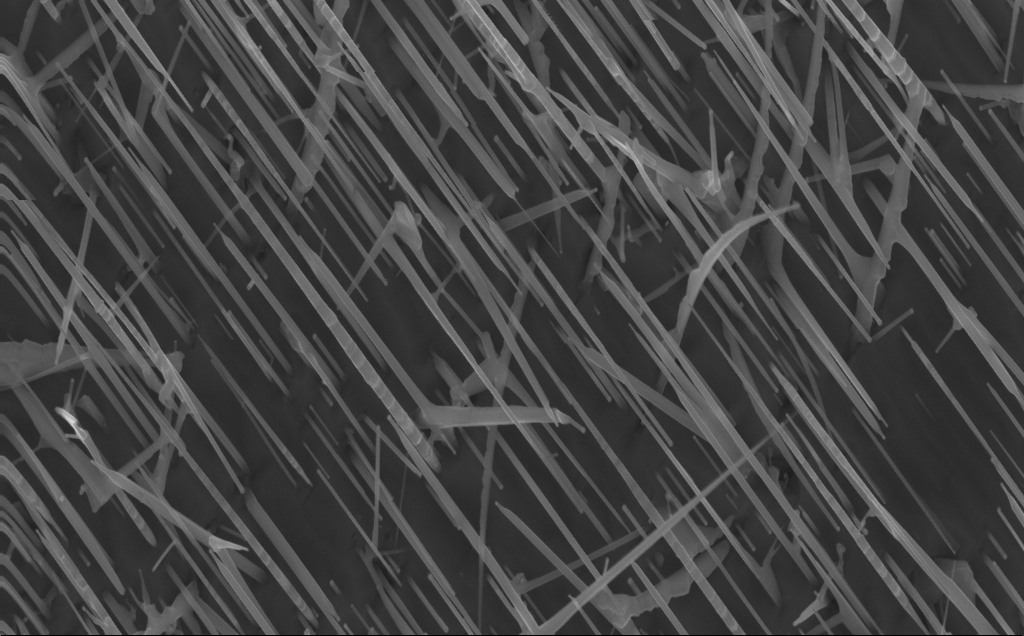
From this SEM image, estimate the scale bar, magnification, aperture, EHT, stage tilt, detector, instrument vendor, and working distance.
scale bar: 1000 nm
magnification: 40 K X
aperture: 30 µm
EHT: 10 kV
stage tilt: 0°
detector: InLens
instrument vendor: Zeiss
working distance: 4 mm